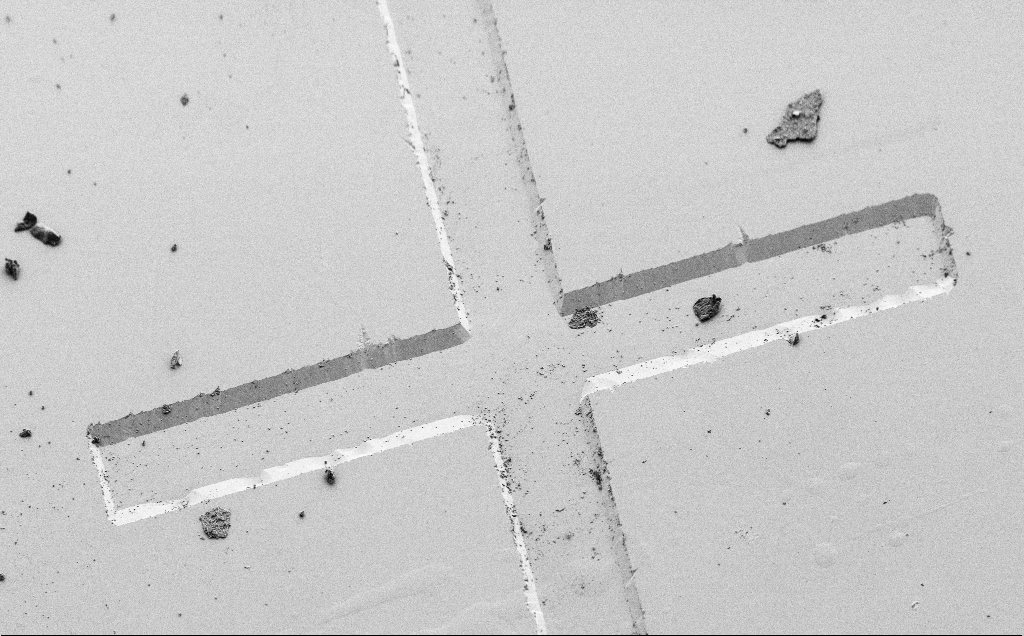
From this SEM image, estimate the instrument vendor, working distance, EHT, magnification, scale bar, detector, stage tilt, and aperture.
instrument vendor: Zeiss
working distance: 9 mm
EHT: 3 kV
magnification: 0.351 K X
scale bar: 100000 nm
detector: SE2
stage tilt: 45°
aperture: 30 µm